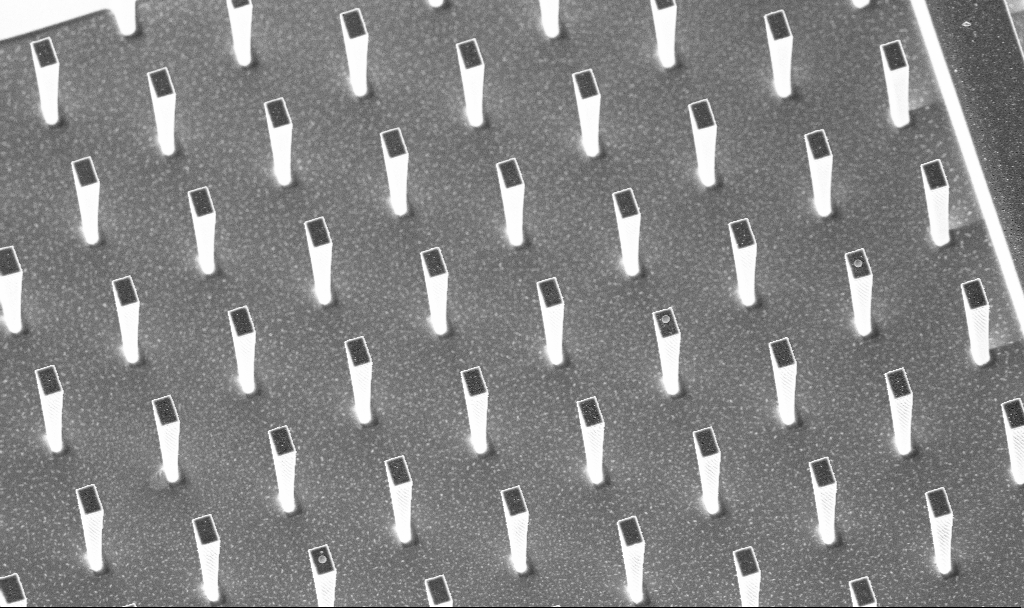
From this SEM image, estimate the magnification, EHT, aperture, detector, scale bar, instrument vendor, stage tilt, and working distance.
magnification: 4.01 K X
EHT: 5 kV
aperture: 30 µm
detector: InLens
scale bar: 10000 nm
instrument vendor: Zeiss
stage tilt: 20°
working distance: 5.2 mm